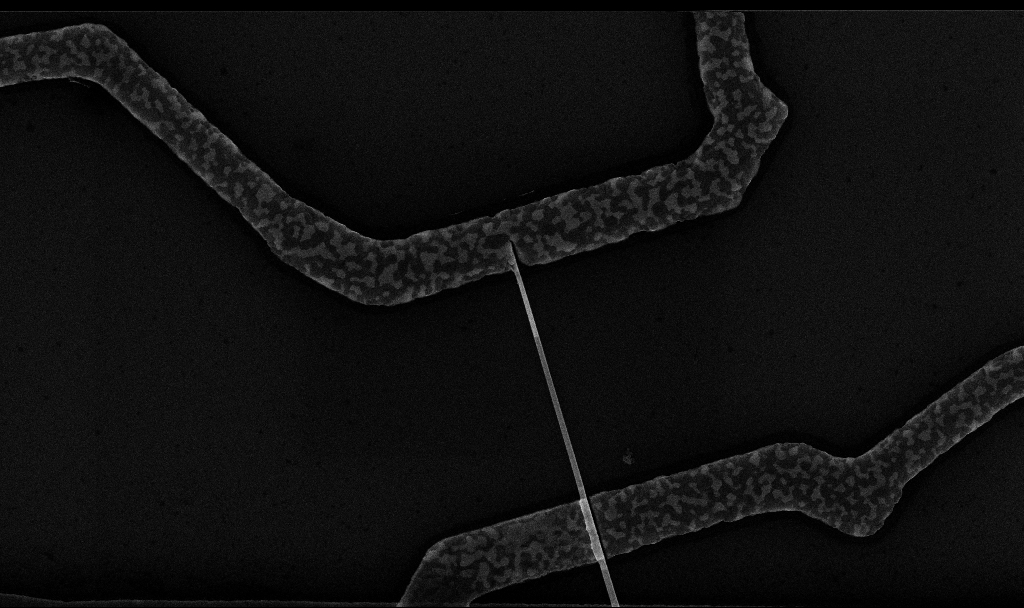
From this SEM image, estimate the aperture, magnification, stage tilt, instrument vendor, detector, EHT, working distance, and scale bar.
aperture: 30 µm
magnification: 26.03 K X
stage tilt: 0°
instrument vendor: Zeiss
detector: InLens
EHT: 10 kV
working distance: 6.7 mm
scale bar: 2000 nm